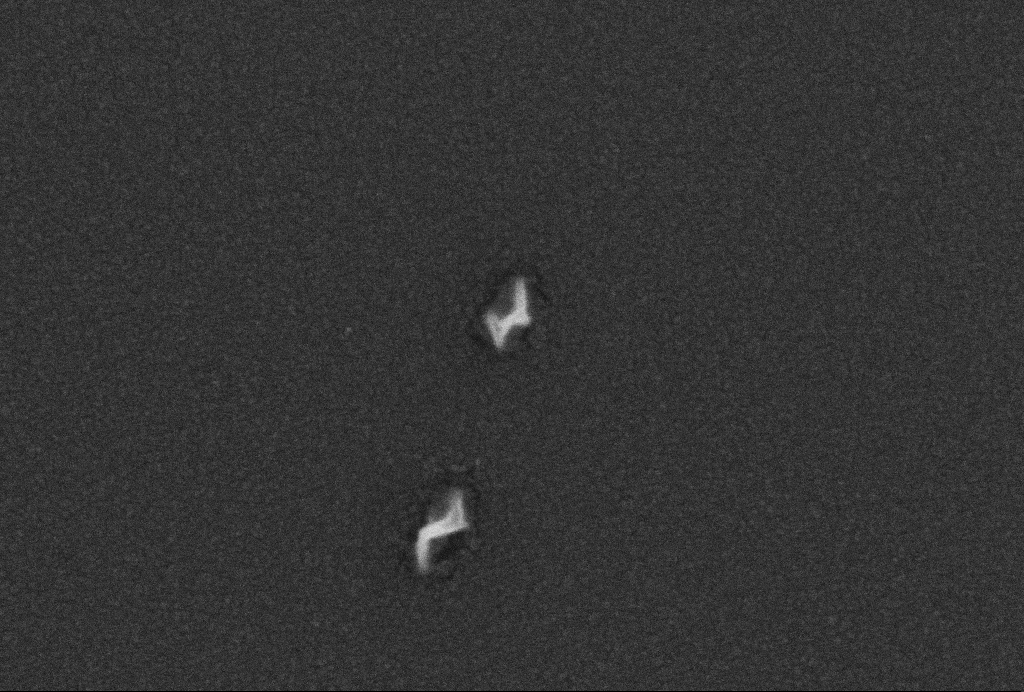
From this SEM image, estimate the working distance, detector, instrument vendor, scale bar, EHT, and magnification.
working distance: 2.6 mm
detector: SE2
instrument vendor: Zeiss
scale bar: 200 nm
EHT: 5 kV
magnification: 250 K X